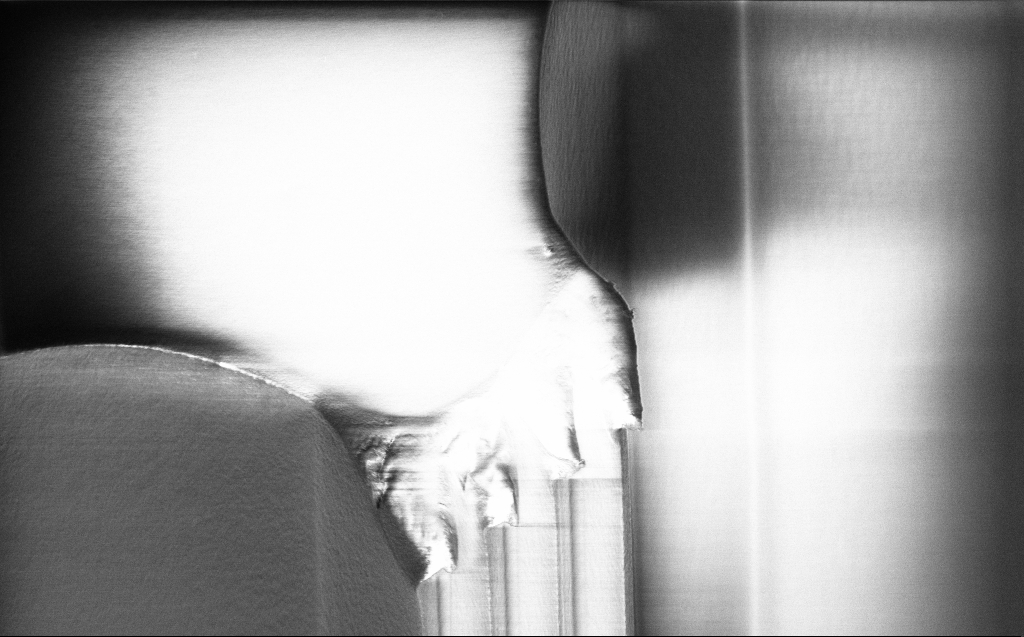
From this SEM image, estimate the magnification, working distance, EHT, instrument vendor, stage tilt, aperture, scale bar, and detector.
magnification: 2.62 K X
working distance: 5 mm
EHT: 1 kV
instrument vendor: Zeiss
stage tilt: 44.6°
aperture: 30 µm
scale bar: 10000 nm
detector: InLens